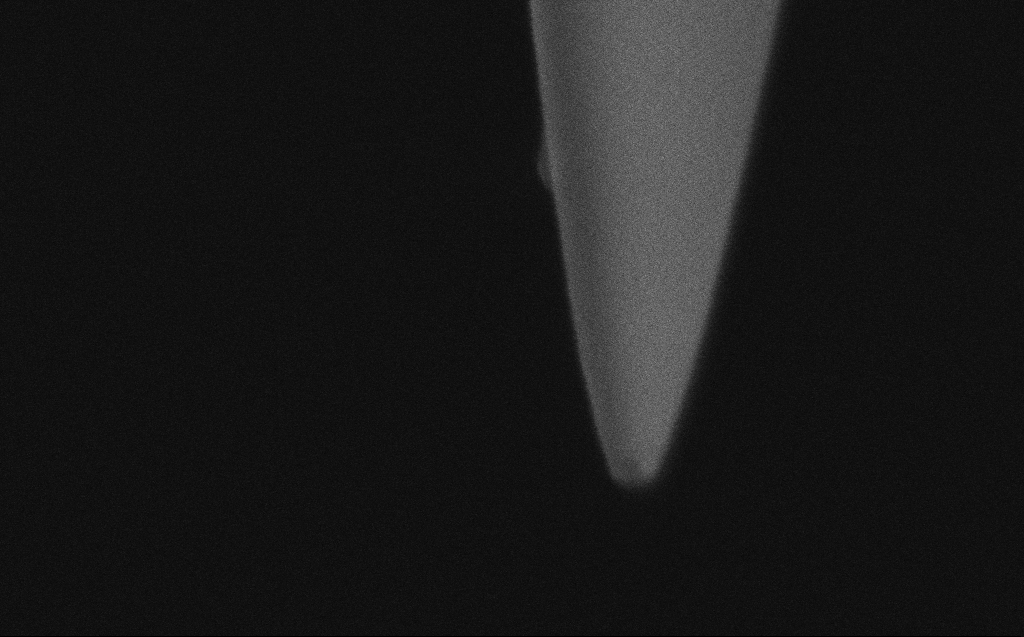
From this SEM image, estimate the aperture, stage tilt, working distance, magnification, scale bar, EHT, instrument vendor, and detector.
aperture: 30 µm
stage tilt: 45.1°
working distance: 3 mm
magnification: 161.01 K X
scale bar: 200 nm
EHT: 0.75 kV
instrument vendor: Zeiss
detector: InLens